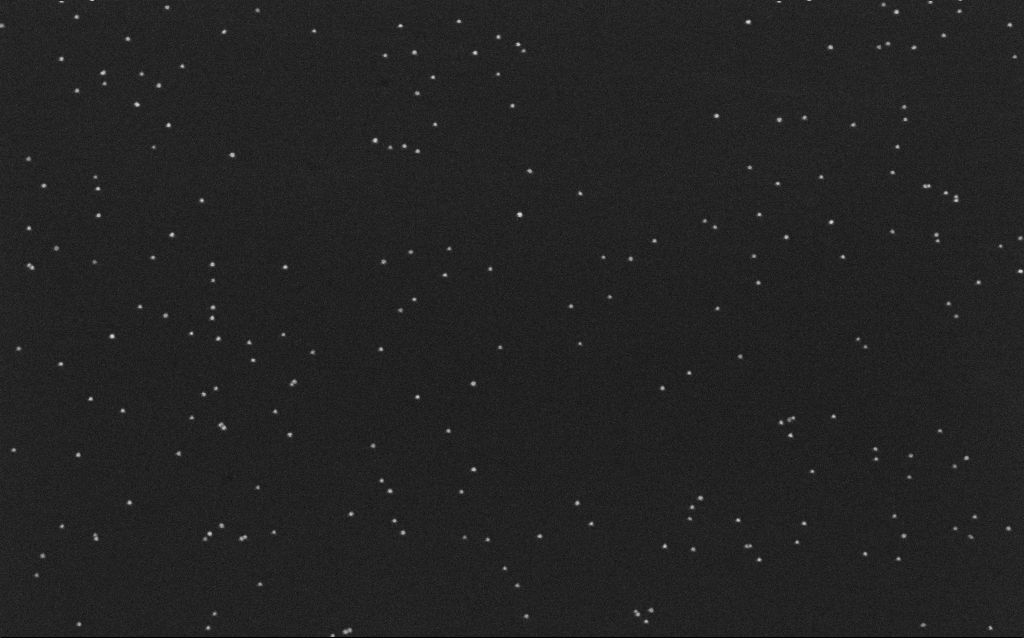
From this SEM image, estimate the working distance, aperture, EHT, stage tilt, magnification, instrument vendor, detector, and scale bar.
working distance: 6.5 mm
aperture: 30 µm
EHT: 10 kV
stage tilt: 0°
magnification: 100 K X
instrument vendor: Zeiss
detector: InLens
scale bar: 200 nm